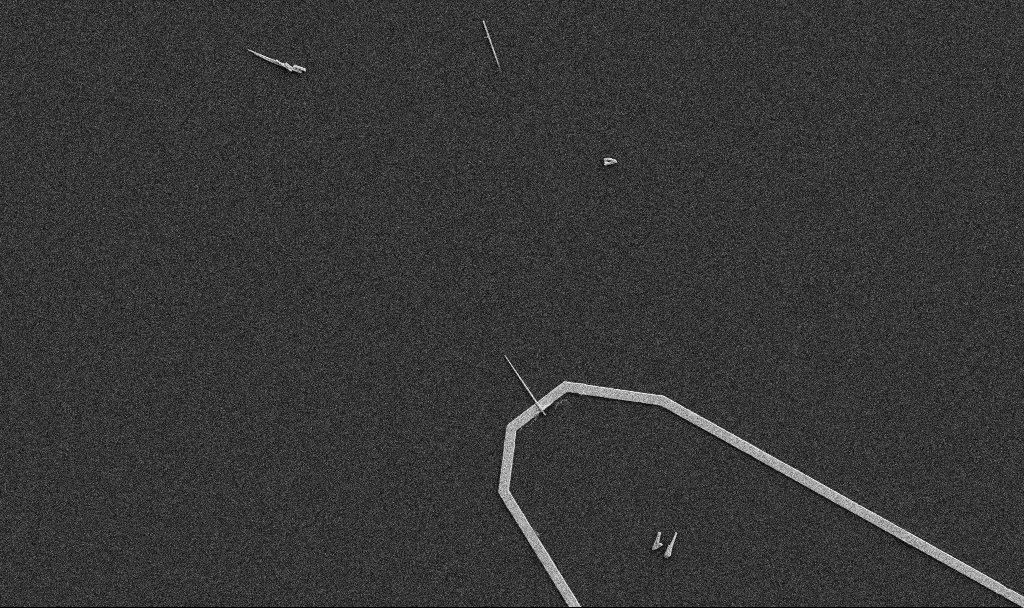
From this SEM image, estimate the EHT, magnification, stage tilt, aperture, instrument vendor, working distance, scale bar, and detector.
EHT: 5 kV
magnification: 5 K X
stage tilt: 0°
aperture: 30 µm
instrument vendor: Zeiss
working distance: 10.7 mm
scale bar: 10000 nm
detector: SE2